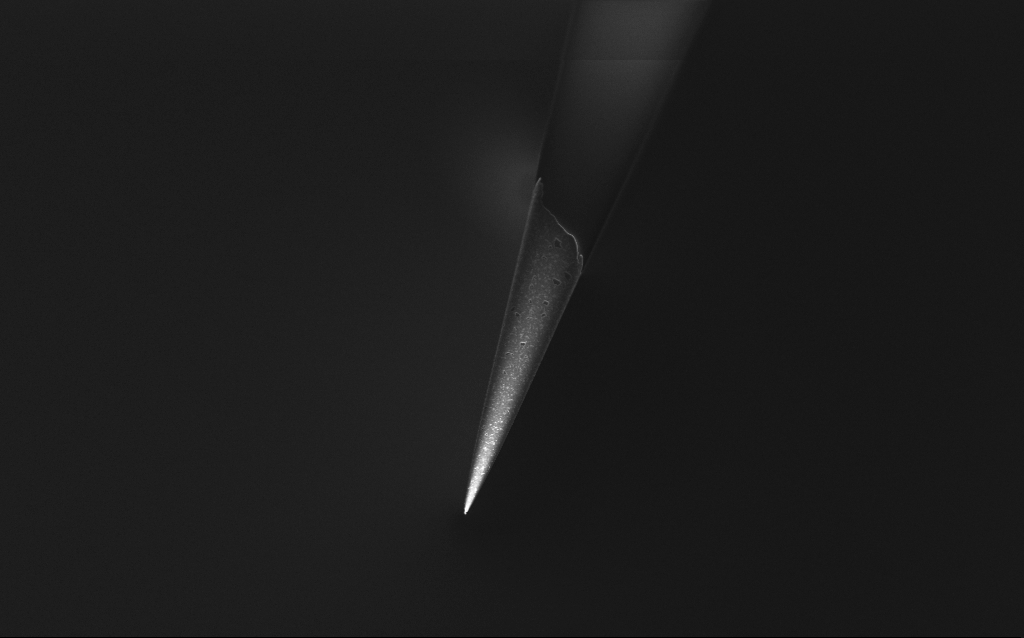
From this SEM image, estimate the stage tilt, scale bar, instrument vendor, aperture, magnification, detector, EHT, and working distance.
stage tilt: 45°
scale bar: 10000 nm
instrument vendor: Zeiss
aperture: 30 µm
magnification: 5 K X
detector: InLens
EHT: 1 kV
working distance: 6 mm